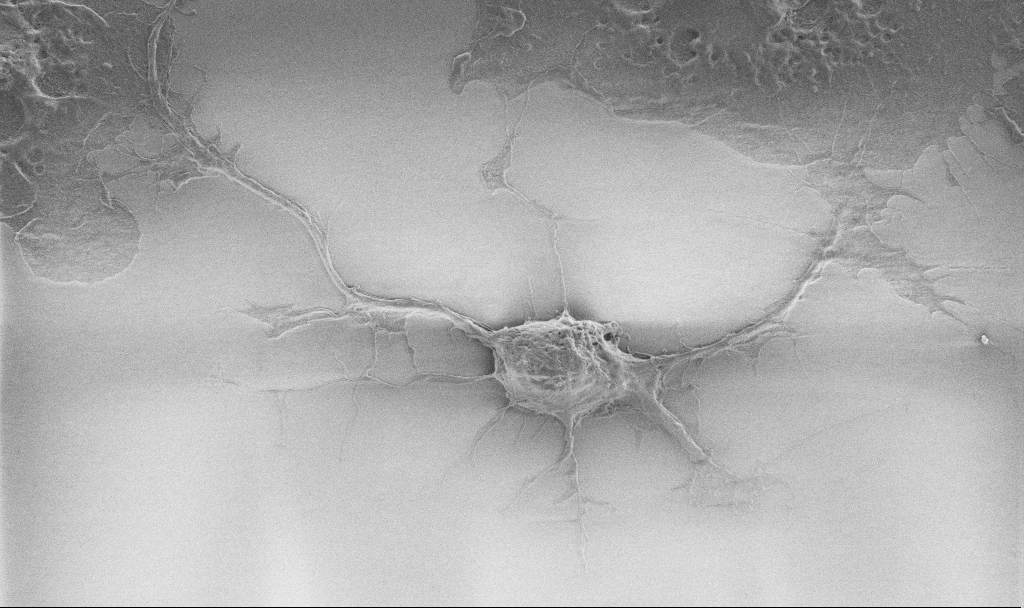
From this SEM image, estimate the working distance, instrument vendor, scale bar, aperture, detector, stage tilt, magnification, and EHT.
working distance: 6.9 mm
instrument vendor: Zeiss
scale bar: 10000 nm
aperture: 30 µm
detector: SE2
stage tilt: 0°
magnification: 5 K X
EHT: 1 kV